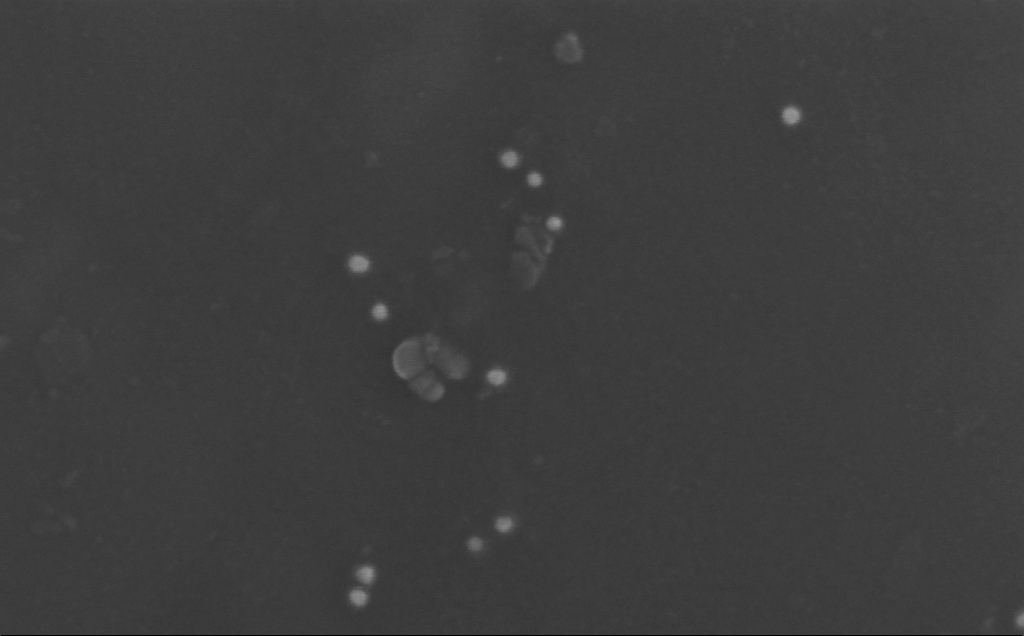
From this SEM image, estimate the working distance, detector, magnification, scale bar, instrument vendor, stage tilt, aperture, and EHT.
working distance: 3 mm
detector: InLens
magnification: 389.08 K X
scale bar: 100 nm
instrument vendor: Zeiss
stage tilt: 0°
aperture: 30 µm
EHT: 10 kV